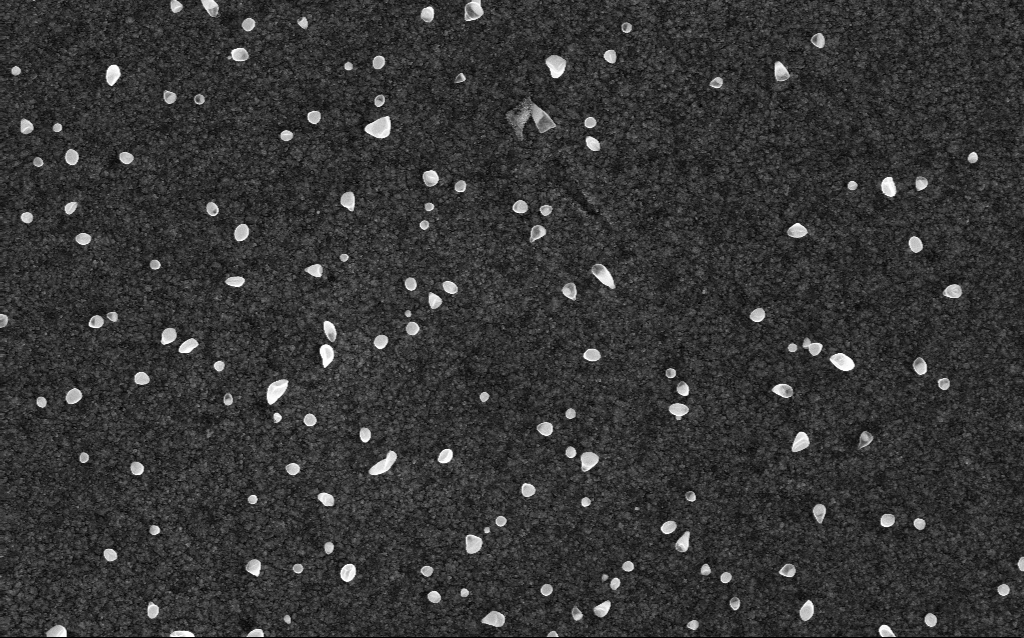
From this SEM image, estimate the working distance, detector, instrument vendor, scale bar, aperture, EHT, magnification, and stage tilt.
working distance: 1.9 mm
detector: InLens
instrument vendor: Zeiss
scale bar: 1000 nm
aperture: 30 µm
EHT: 5 kV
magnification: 50 K X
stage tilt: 0°